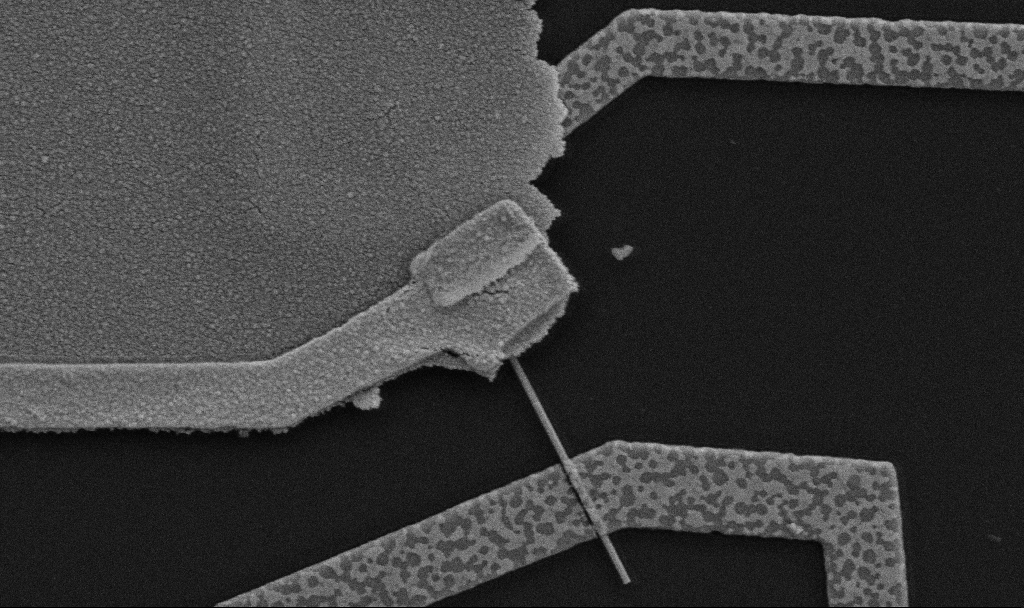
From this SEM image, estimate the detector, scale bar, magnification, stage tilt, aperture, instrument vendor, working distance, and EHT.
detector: SE2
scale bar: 1000 nm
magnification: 30 K X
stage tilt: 0°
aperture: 30 µm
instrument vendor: Zeiss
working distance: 10.7 mm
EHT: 5 kV